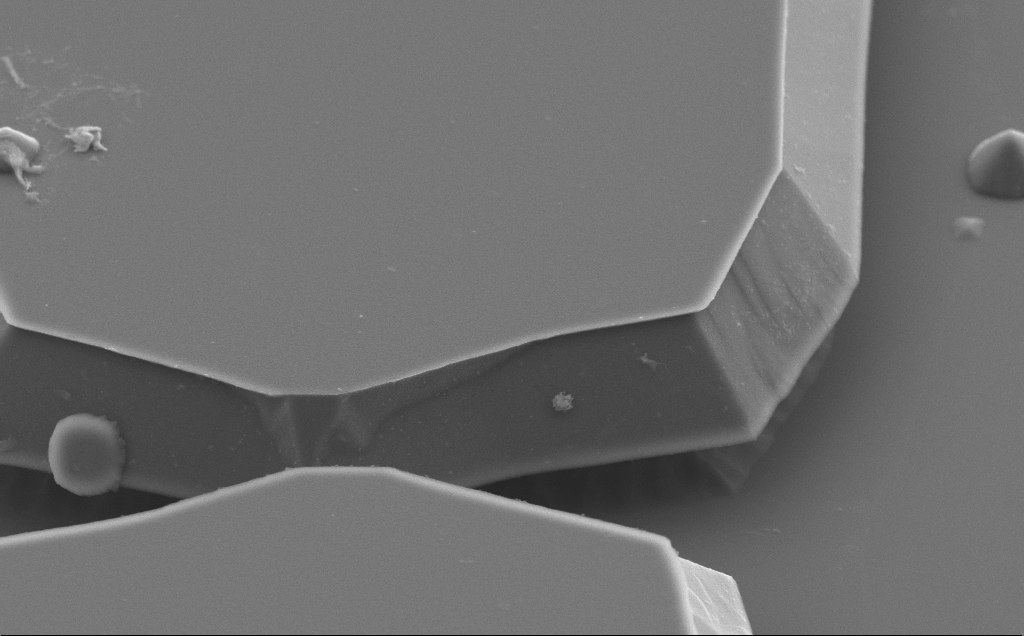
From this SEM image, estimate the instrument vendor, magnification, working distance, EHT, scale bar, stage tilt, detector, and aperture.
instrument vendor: Zeiss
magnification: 16.61 K X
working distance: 10 mm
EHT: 5 kV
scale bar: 2000 nm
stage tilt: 50°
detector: SE2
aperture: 30 µm